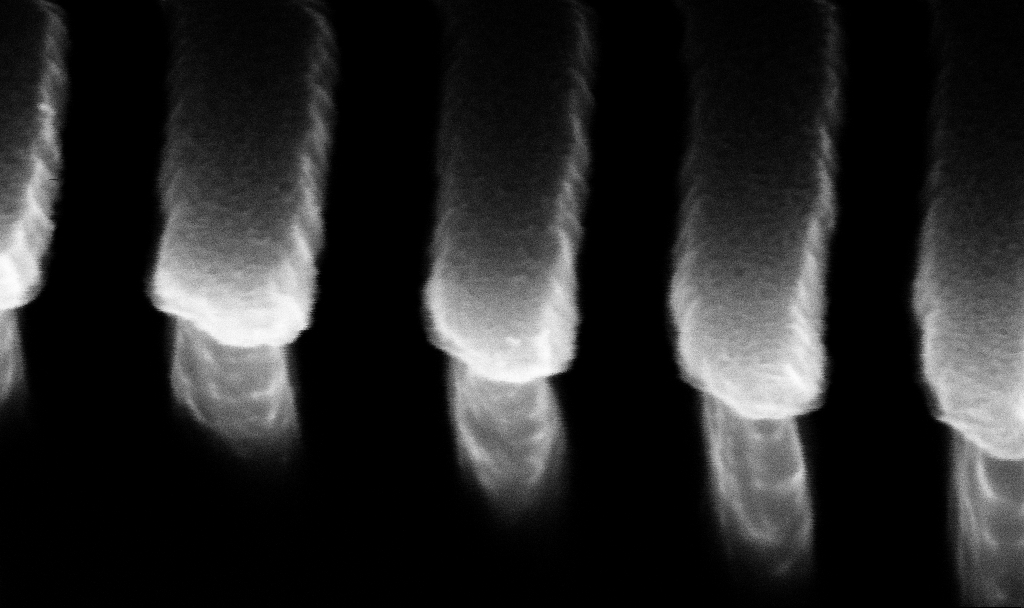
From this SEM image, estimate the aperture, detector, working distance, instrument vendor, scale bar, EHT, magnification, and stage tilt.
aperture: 30 µm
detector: InLens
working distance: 8.8 mm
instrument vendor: Zeiss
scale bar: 100 nm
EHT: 5 kV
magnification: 362.95 K X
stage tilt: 45°